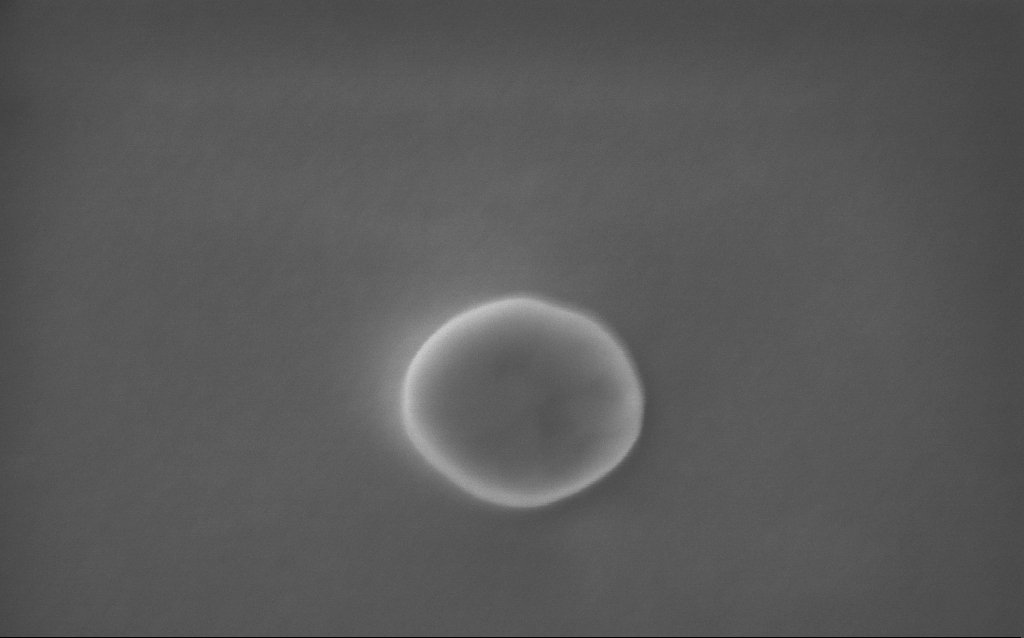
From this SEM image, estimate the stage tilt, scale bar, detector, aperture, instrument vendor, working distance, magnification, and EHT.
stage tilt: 0°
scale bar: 200 nm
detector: InLens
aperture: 30 µm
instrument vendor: Zeiss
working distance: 2 mm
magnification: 178 K X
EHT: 5 kV